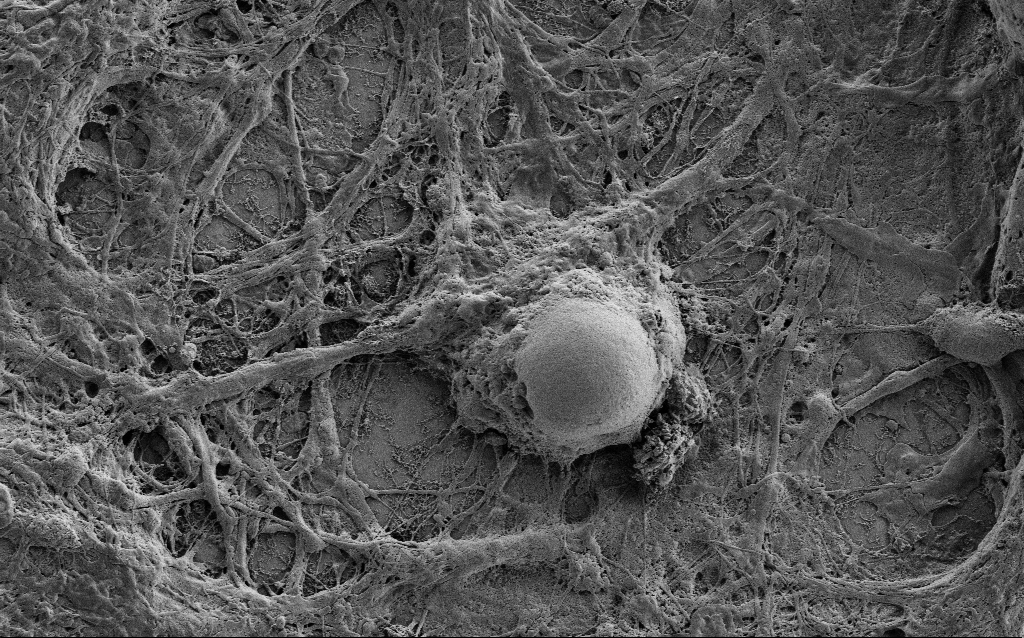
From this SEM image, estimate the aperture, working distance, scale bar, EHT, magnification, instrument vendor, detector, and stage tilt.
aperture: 30 µm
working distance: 7 mm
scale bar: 2000 nm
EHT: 1 kV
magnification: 7.5 K X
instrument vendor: Zeiss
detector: SE2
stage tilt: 0°